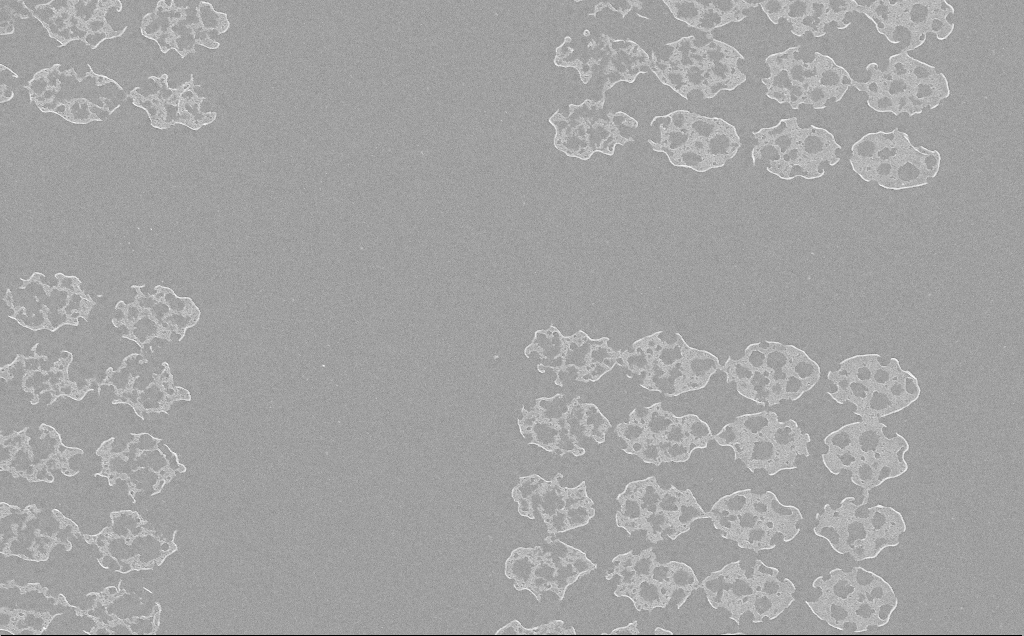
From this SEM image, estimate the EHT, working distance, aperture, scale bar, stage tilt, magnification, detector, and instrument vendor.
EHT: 5 kV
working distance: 6 mm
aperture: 30 µm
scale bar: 10000 nm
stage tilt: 0°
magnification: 3.81 K X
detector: InLens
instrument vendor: Zeiss